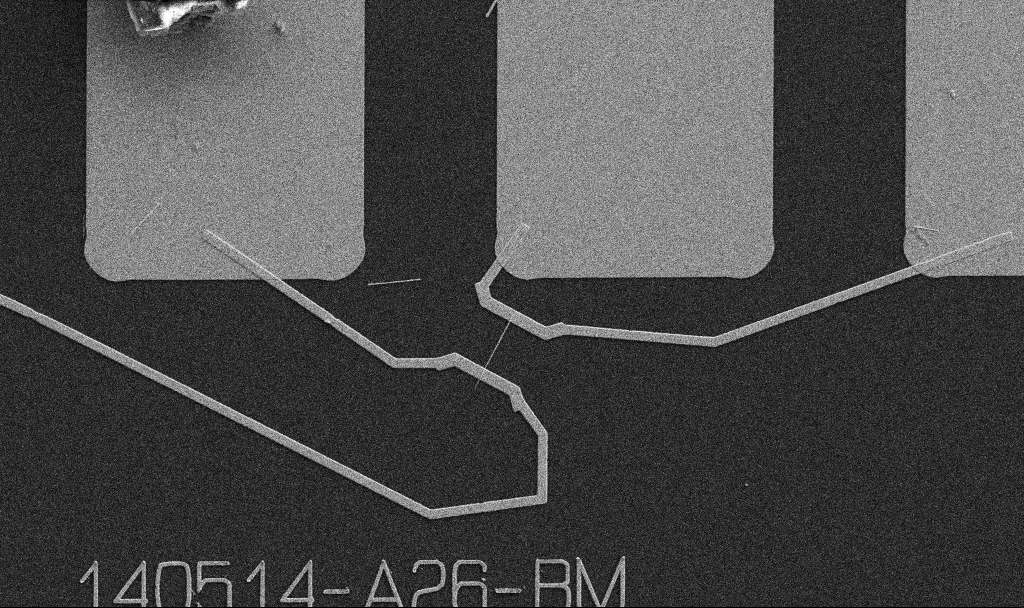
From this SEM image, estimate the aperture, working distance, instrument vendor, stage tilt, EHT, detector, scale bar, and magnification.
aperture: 30 µm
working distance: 10.7 mm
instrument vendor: Zeiss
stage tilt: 0°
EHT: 5 kV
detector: SE2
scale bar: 10000 nm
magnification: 5 K X